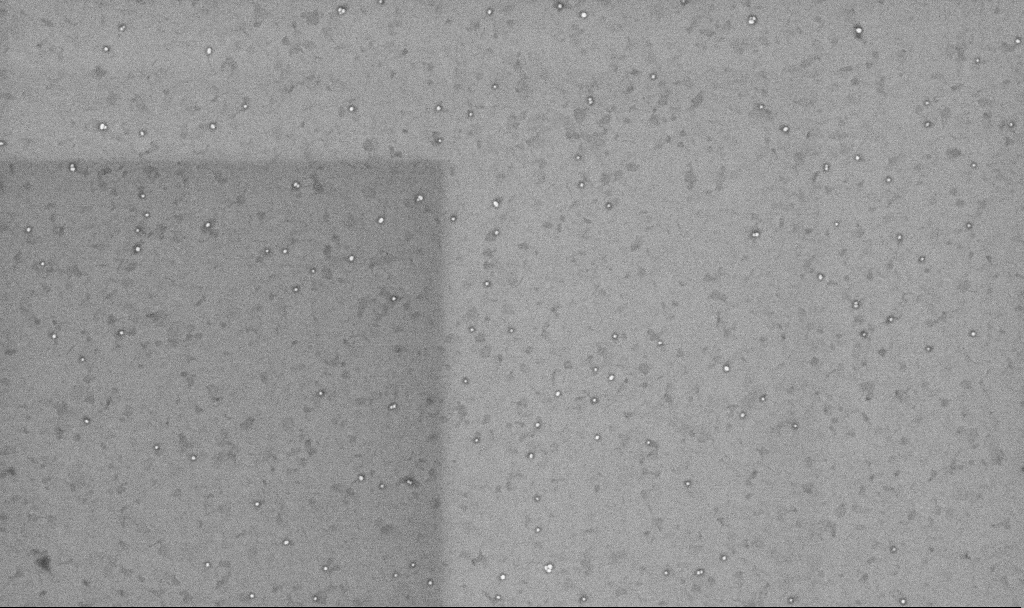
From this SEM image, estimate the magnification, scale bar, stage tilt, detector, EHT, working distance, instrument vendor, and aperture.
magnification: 50.38 K X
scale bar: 1000 nm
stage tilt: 0°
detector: InLens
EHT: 10 kV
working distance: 3.3 mm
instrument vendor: Zeiss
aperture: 30 µm